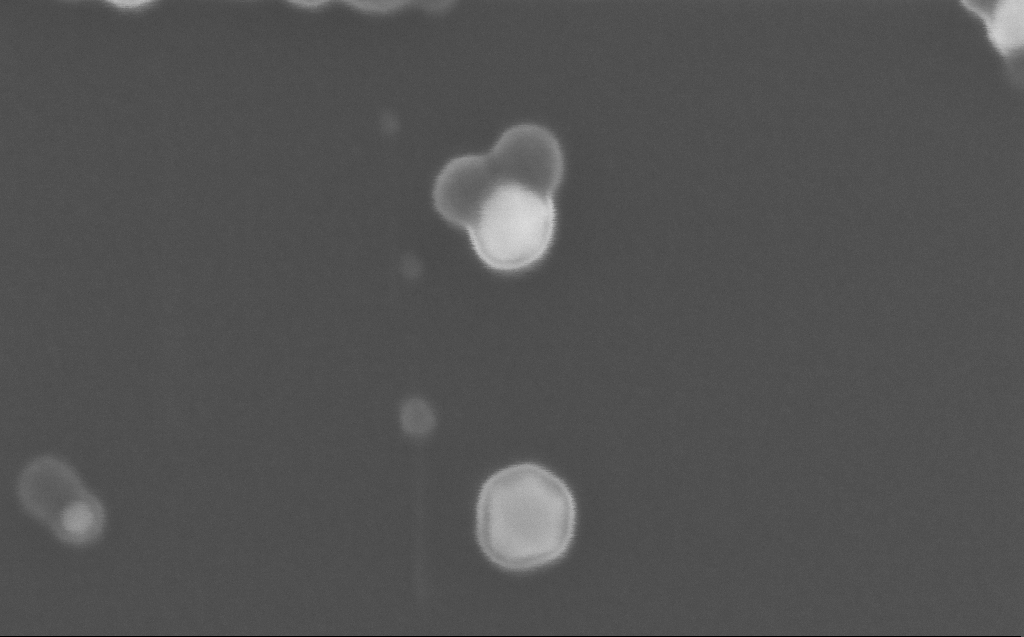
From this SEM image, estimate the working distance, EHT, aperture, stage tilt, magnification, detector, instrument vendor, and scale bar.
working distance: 3 mm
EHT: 10 kV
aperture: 30 µm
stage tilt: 0°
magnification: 600 K X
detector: InLens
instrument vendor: Zeiss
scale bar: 100 nm